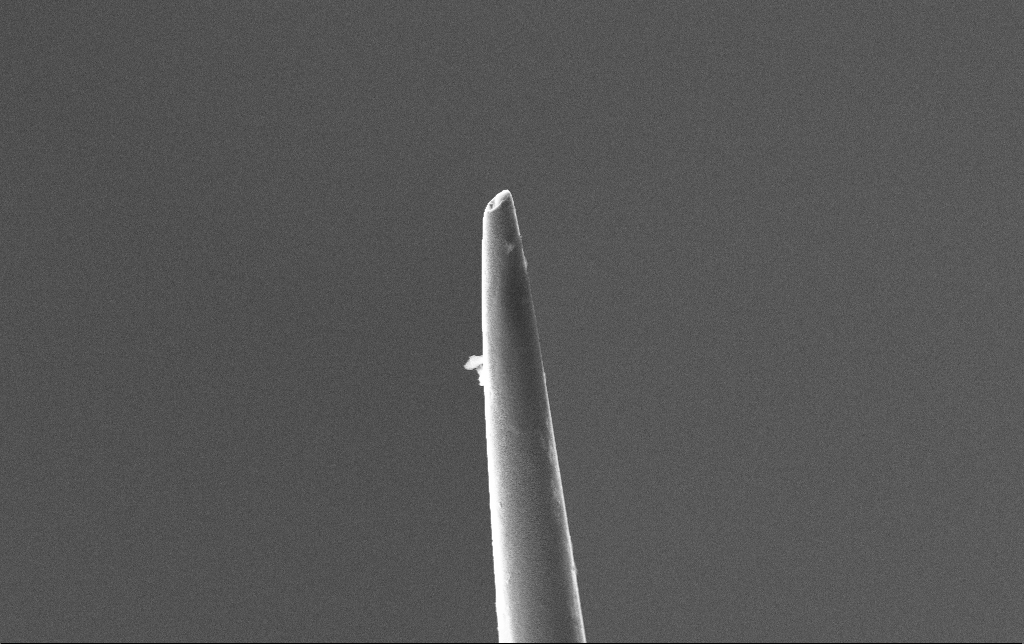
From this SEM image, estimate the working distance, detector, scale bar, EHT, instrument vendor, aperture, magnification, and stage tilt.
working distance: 5.3 mm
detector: SE2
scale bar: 10000 nm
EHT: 10 kV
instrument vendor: Zeiss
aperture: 30 µm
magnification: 4.4 K X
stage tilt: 27.9°